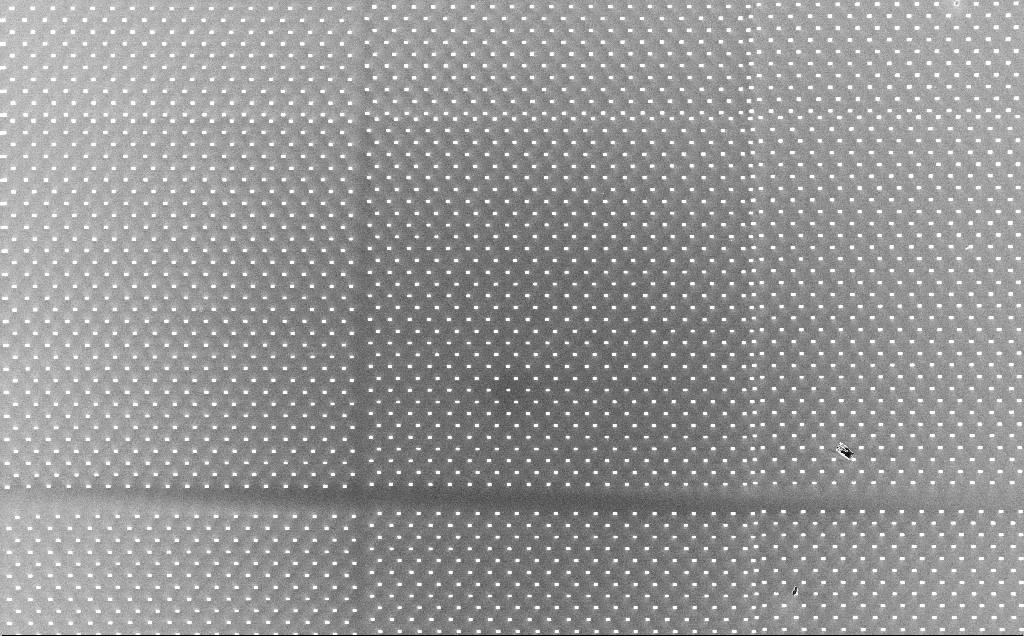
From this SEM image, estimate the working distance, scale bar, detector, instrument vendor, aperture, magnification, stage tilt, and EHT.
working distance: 4 mm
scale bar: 100000 nm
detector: InLens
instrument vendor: Zeiss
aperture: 30 µm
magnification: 0.483 K X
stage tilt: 0°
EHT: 3 kV